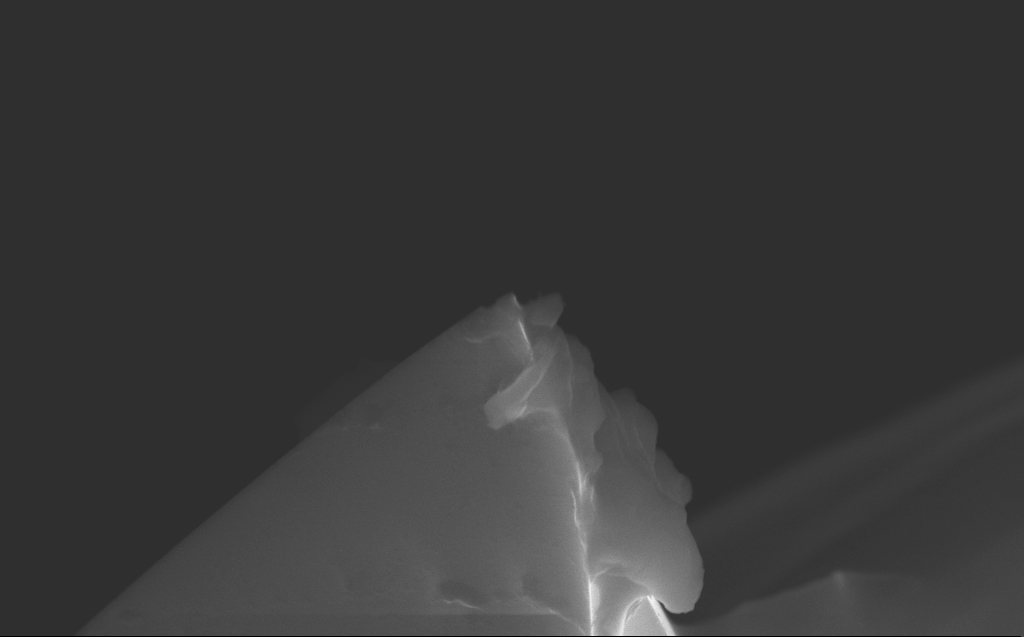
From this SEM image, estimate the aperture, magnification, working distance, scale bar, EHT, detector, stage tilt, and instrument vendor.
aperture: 30 µm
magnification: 39.38 K X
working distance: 8 mm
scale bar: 1000 nm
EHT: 10 kV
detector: InLens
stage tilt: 35.7°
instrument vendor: Zeiss